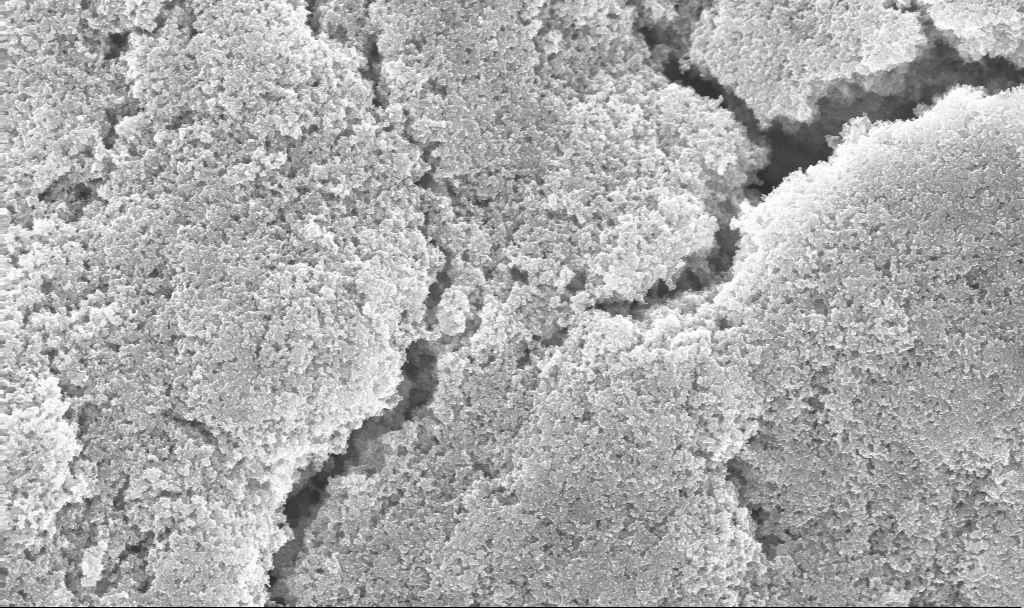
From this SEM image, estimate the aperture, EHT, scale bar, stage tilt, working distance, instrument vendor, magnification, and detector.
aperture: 30 µm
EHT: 10 kV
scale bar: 1000 nm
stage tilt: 0°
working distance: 2.7 mm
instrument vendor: Zeiss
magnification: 24.02 K X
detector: InLens